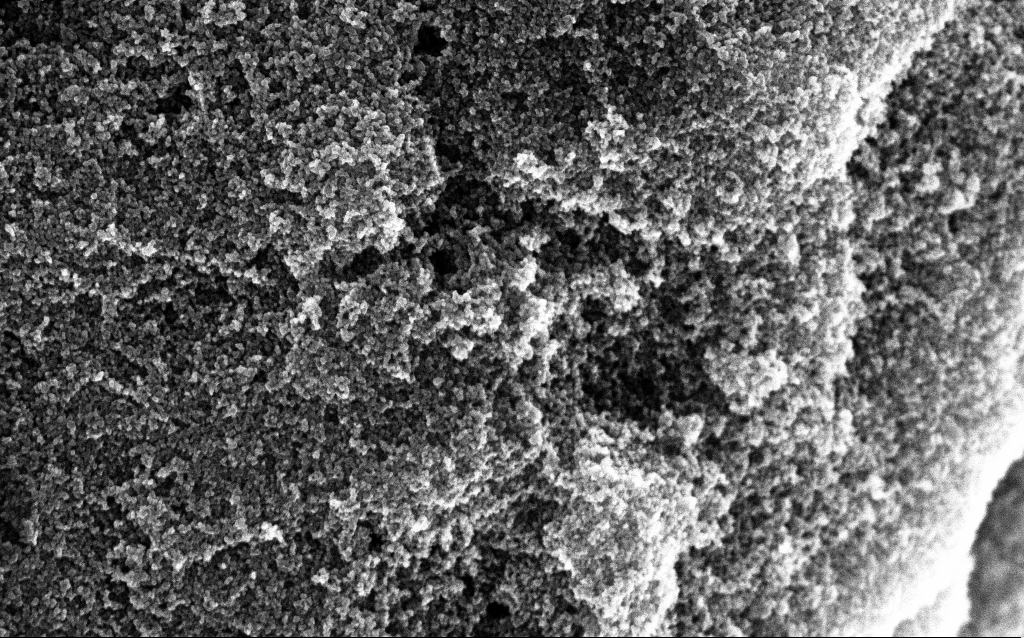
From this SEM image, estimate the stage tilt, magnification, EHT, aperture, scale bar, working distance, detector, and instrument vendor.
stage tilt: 0°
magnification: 65.04 K X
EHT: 10 kV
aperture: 30 µm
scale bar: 1000 nm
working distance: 2.8 mm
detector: InLens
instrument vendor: Zeiss